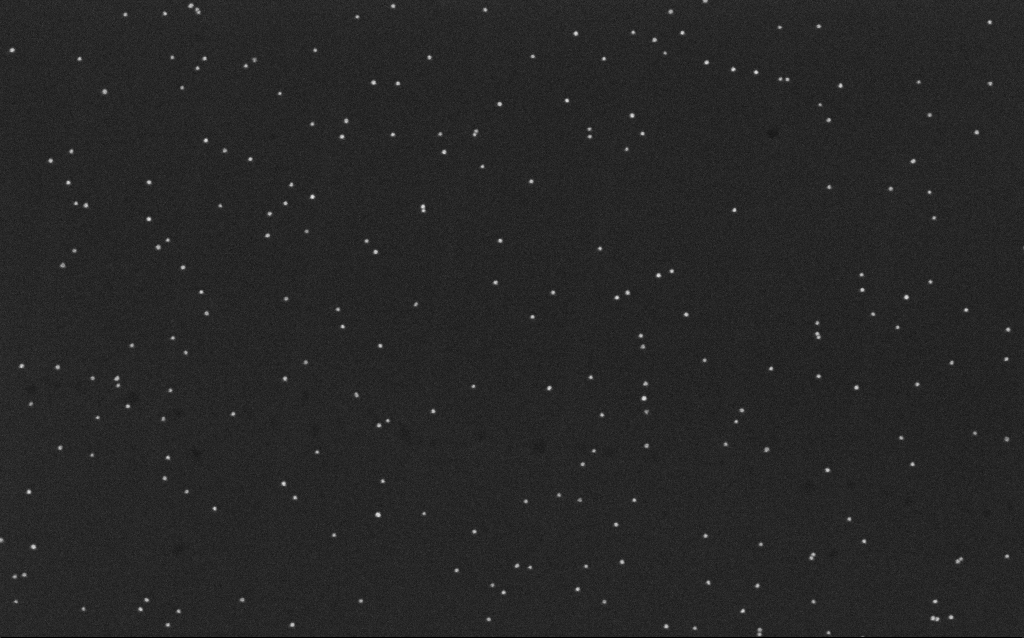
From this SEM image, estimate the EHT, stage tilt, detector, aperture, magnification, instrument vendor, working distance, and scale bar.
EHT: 10 kV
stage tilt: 0°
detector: InLens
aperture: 30 µm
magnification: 100 K X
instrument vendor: Zeiss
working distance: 6.5 mm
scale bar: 200 nm